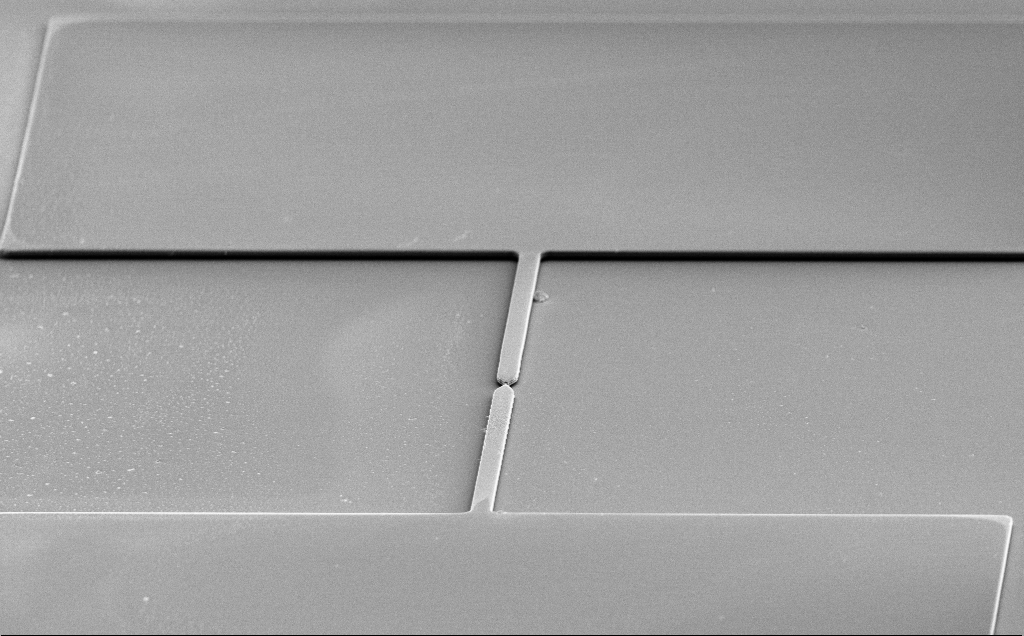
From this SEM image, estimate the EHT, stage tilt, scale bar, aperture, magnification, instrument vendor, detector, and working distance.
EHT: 8 kV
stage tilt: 70°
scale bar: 20000 nm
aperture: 30 µm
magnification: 0.938 K X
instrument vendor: Zeiss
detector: SE2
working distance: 12 mm